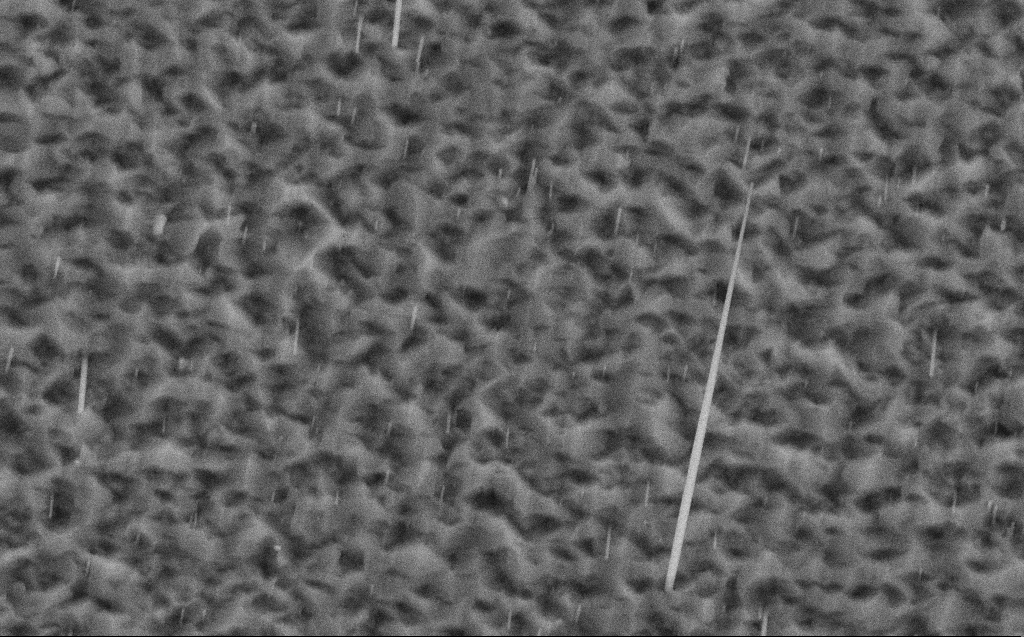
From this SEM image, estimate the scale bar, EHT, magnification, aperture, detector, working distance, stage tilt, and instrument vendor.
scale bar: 2000 nm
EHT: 10 kV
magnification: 26.42 K X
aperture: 30 µm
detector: SE2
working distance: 3 mm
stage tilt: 0°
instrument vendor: Zeiss